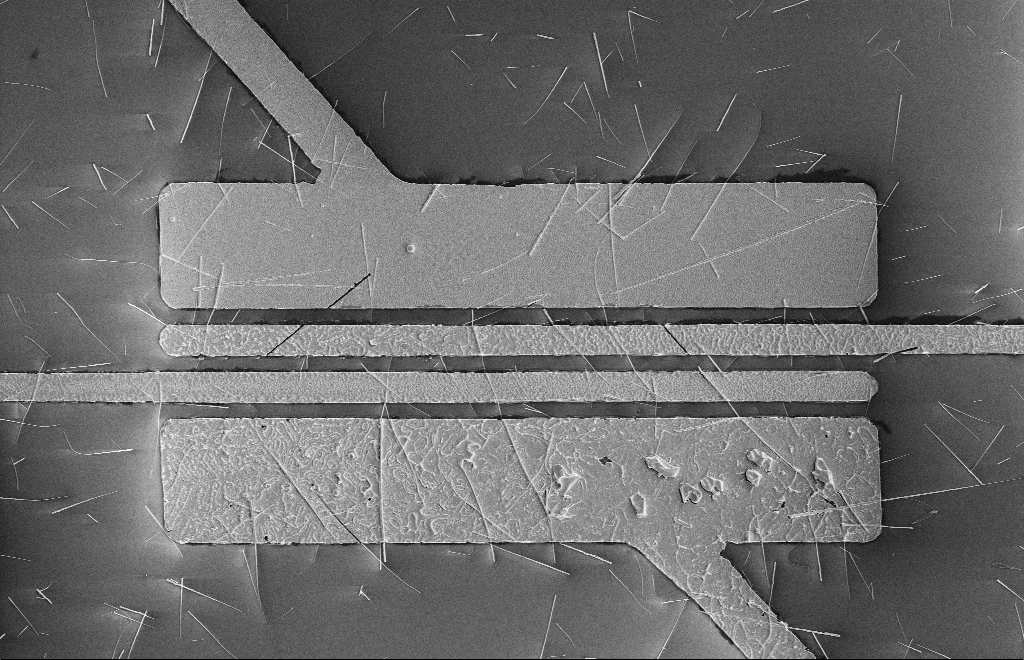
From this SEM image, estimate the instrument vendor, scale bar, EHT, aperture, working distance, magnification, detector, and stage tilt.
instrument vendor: Zeiss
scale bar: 10000 nm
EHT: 5 kV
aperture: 10 µm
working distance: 16 mm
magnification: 4.34 K X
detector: SE2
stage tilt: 0°